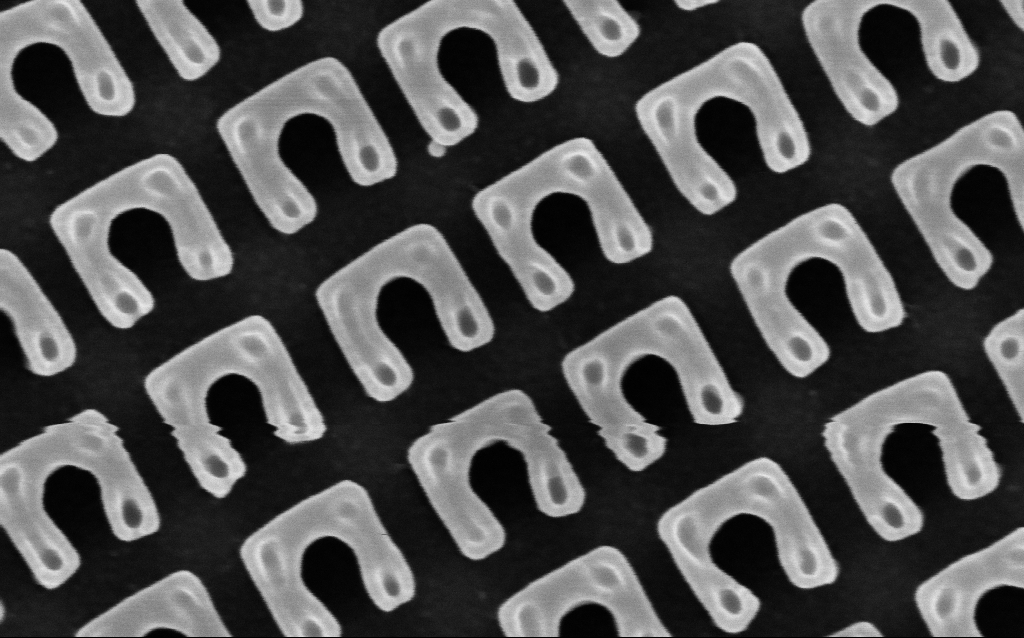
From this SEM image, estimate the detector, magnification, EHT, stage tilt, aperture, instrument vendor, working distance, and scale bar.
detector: InLens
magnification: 150.76 K X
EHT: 3 kV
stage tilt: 0°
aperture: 30 µm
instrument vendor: Zeiss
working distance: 4.6 mm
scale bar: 200 nm